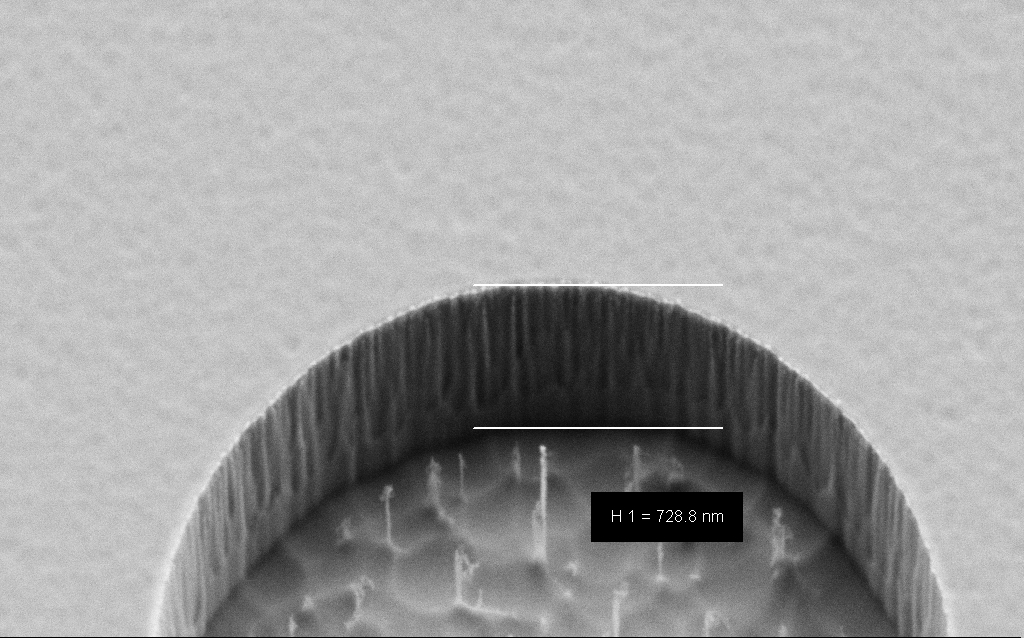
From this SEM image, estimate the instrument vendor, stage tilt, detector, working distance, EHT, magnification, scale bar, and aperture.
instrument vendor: Zeiss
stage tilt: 45°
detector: SE2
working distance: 6 mm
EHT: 2 kV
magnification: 72.05 K X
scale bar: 1000 nm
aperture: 30 µm